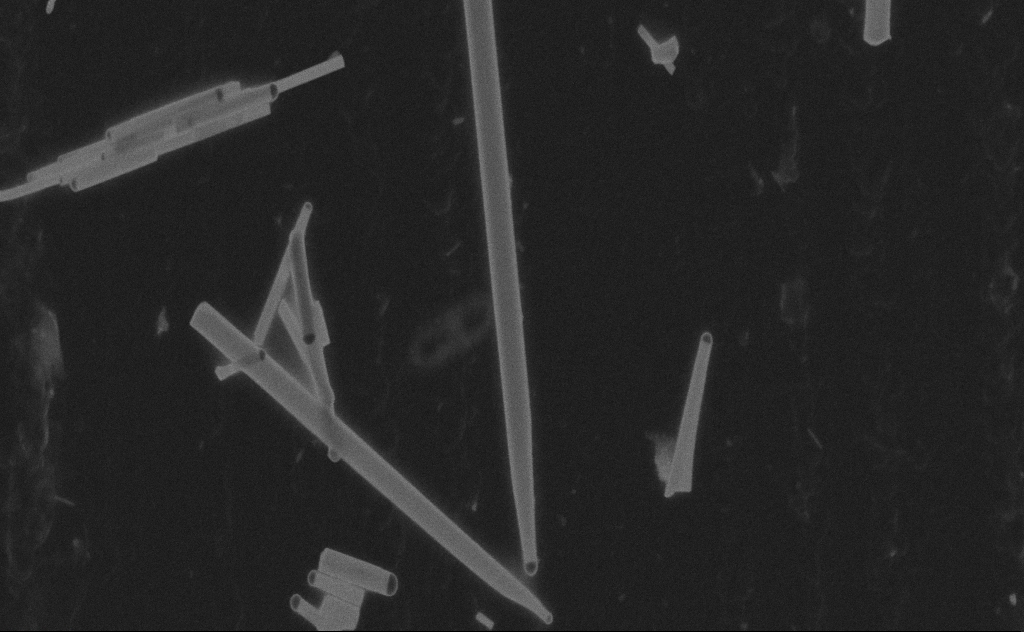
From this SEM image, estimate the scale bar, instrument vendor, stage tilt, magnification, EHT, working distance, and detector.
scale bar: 200 nm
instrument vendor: Zeiss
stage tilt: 0°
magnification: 83.33 K X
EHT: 20 kV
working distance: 9 mm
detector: SE2